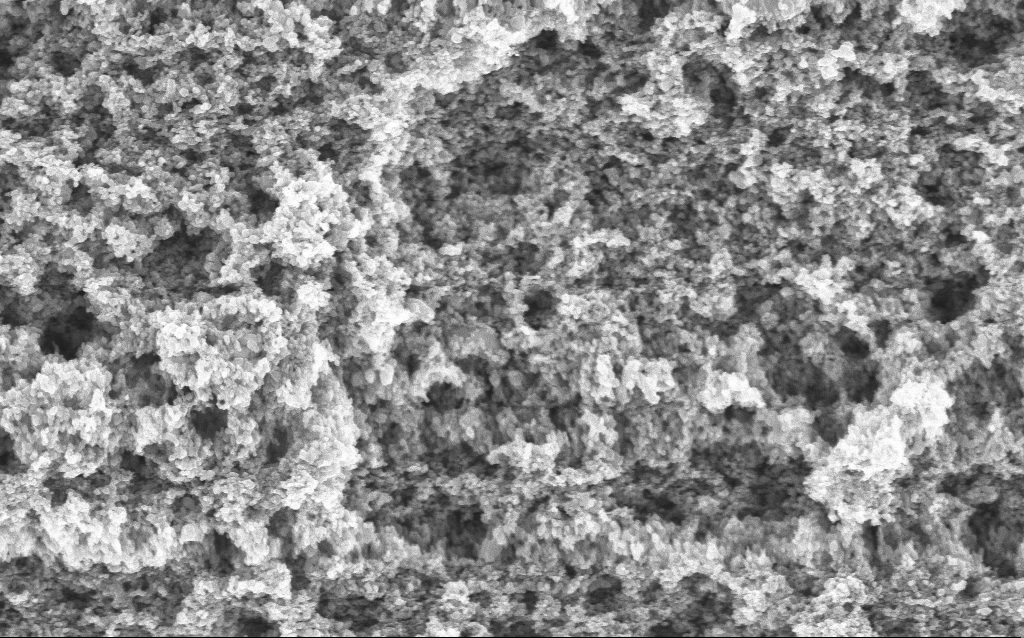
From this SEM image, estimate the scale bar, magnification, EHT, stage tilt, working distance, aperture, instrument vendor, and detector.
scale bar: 1000 nm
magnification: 65.04 K X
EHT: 5 kV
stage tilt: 0°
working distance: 4.4 mm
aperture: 30 µm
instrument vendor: Zeiss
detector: InLens